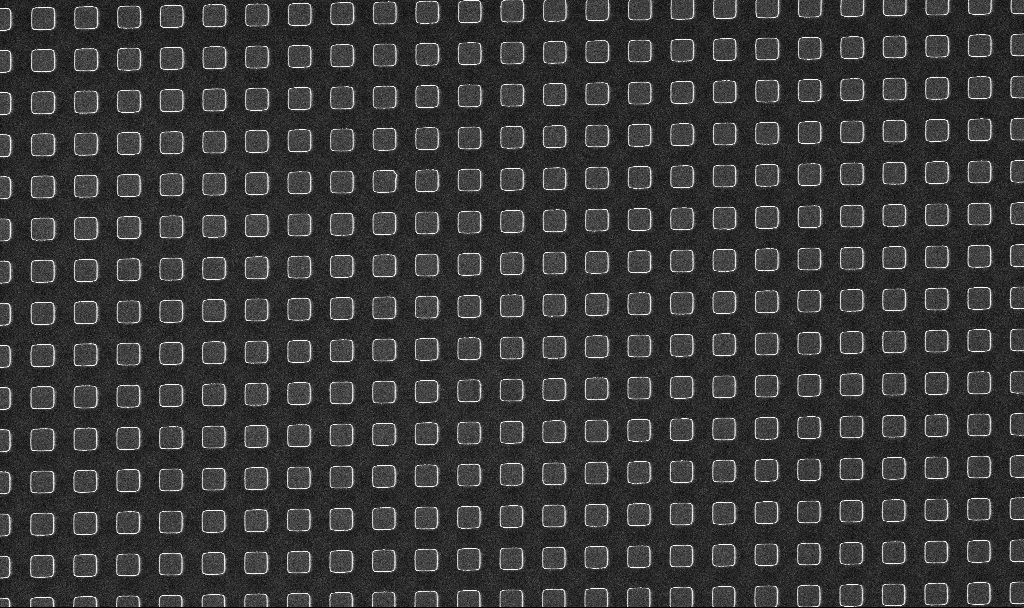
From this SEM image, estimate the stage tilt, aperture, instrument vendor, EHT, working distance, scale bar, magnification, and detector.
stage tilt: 0°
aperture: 30 µm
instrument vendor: Zeiss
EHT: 3 kV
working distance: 7.8 mm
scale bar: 20000 nm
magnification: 1.95 K X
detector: InLens